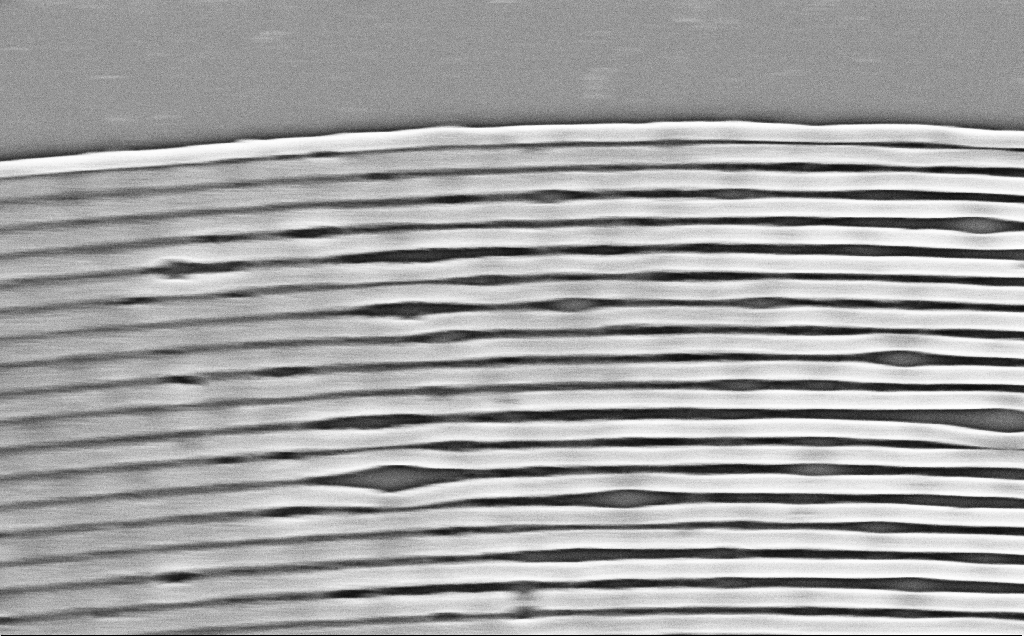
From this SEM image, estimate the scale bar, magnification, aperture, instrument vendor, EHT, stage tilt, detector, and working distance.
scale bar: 2000 nm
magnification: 33.09 K X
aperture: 30 µm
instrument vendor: Zeiss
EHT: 5 kV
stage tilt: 0°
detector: InLens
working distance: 7 mm